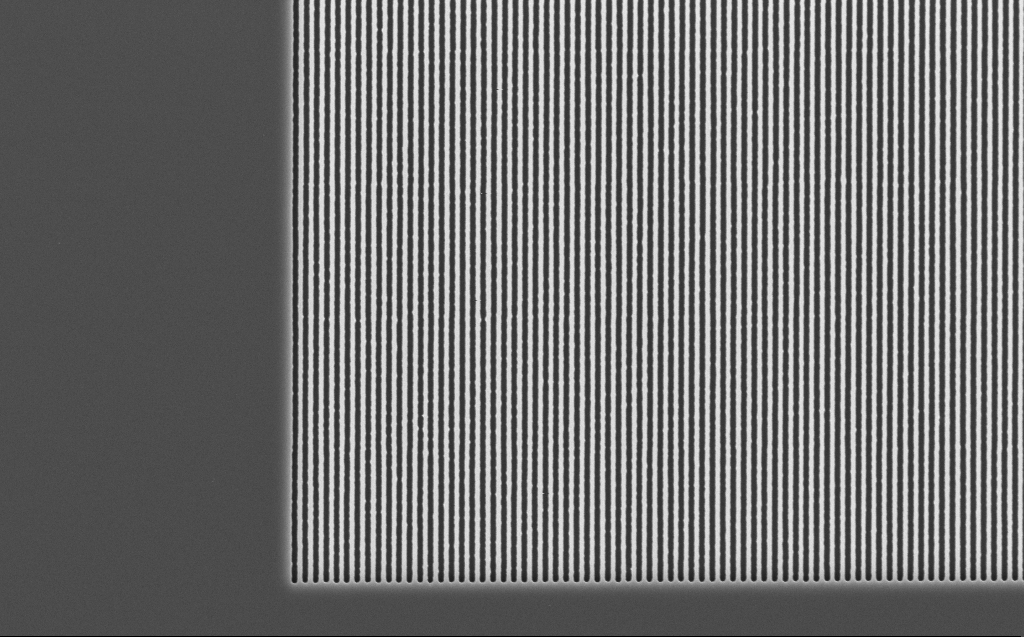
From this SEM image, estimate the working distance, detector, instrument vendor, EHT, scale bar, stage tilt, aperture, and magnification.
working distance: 7 mm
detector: InLens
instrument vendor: Zeiss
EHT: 5 kV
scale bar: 2000 nm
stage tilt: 0°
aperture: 30 µm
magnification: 19.21 K X